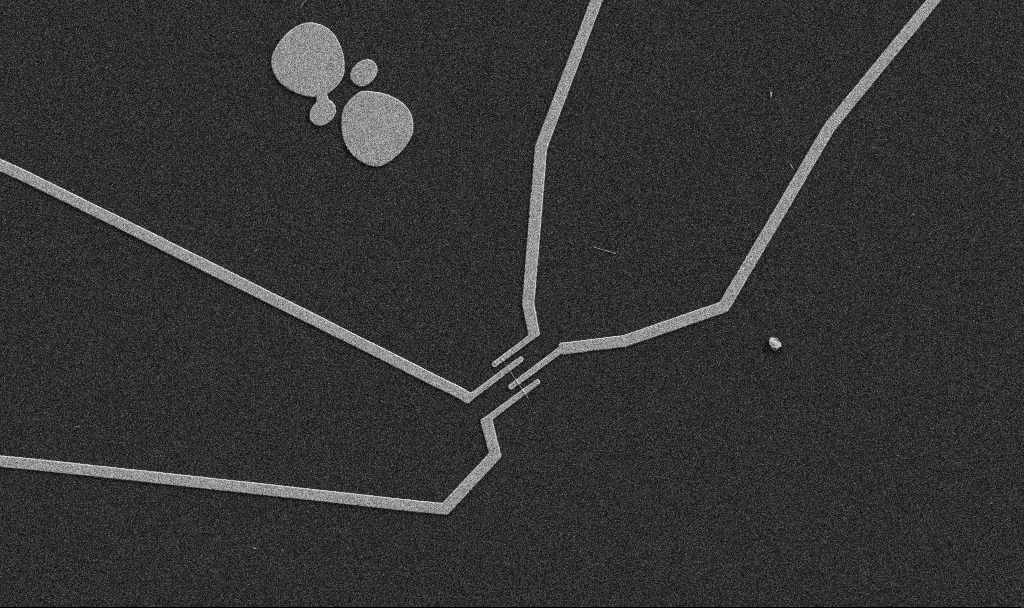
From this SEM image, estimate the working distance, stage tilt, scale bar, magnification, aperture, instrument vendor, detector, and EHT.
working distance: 10.7 mm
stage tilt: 0°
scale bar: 10000 nm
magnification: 5 K X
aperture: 30 µm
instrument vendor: Zeiss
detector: SE2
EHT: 5 kV